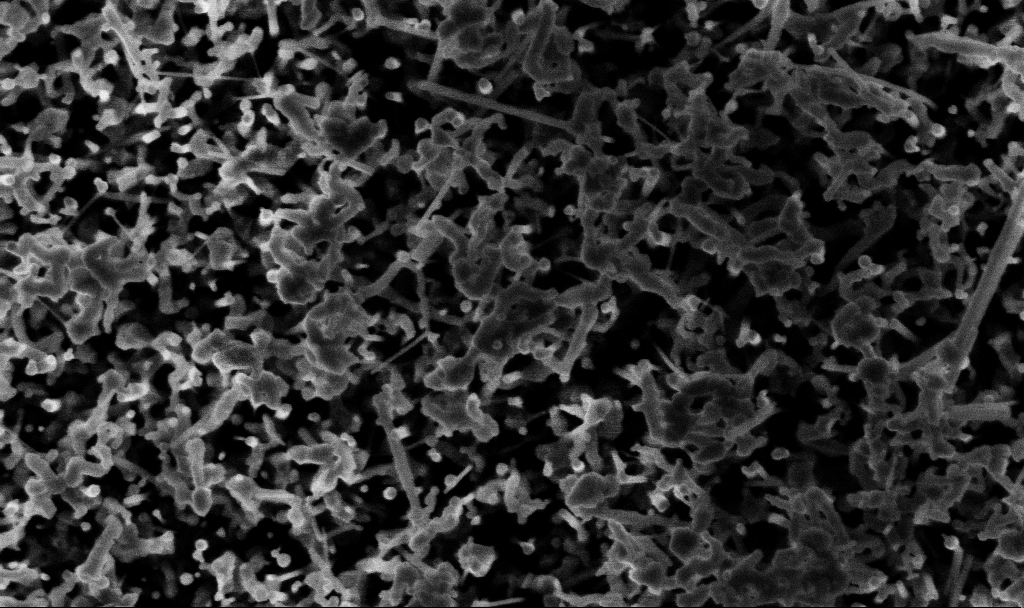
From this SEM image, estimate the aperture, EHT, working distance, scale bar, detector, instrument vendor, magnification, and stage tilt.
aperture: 30 µm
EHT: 3 kV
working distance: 3.1 mm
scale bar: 200 nm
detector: InLens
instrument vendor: Zeiss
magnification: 88.79 K X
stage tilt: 0°